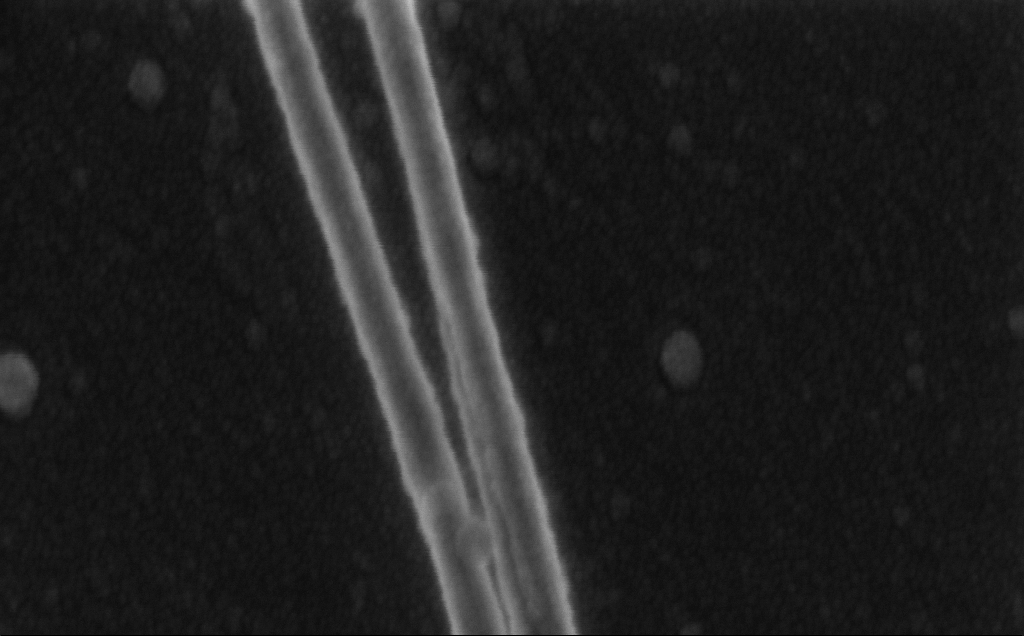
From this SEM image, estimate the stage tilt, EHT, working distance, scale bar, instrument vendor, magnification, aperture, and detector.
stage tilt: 0.8°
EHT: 3 kV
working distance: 6 mm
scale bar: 200 nm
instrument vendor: Zeiss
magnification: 181.47 K X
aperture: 30 µm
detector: InLens